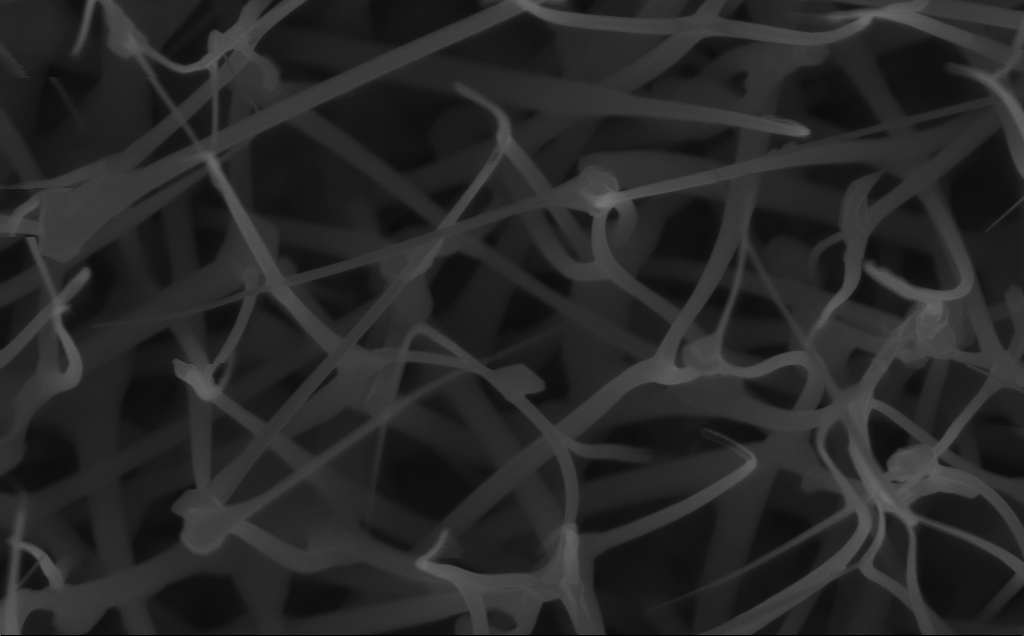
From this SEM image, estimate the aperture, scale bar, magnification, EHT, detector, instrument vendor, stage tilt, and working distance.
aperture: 30 µm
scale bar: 200 nm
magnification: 80 K X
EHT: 10 kV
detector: InLens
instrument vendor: Zeiss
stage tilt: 0°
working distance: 6 mm